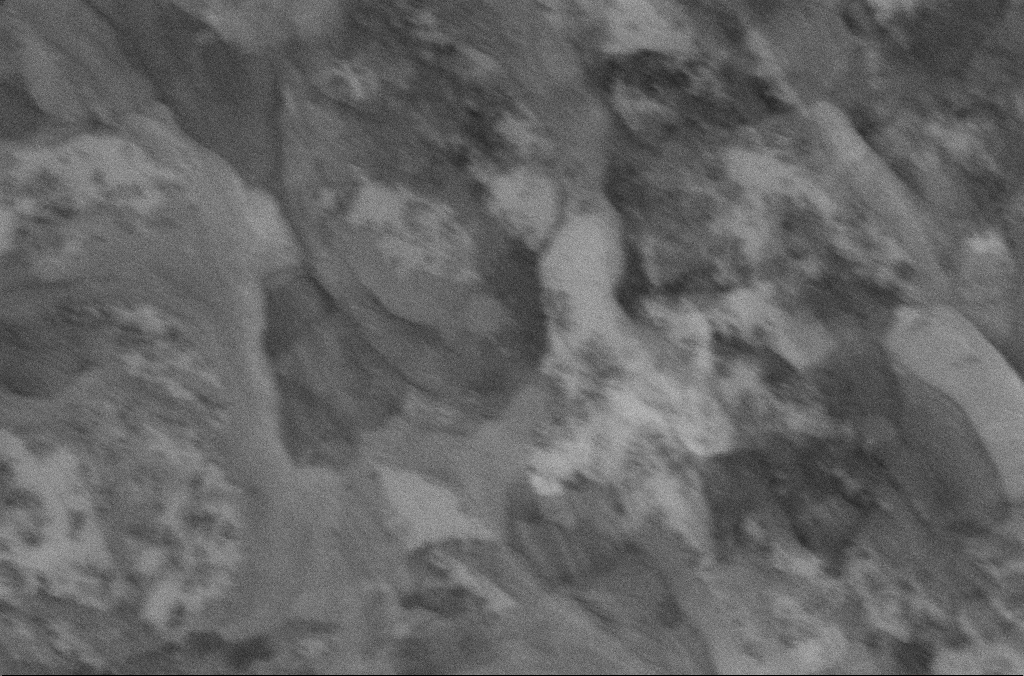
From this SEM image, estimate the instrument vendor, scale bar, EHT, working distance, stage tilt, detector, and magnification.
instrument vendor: Zeiss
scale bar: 200 nm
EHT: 5 kV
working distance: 6.7 mm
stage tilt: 0°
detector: InLens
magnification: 100 K X